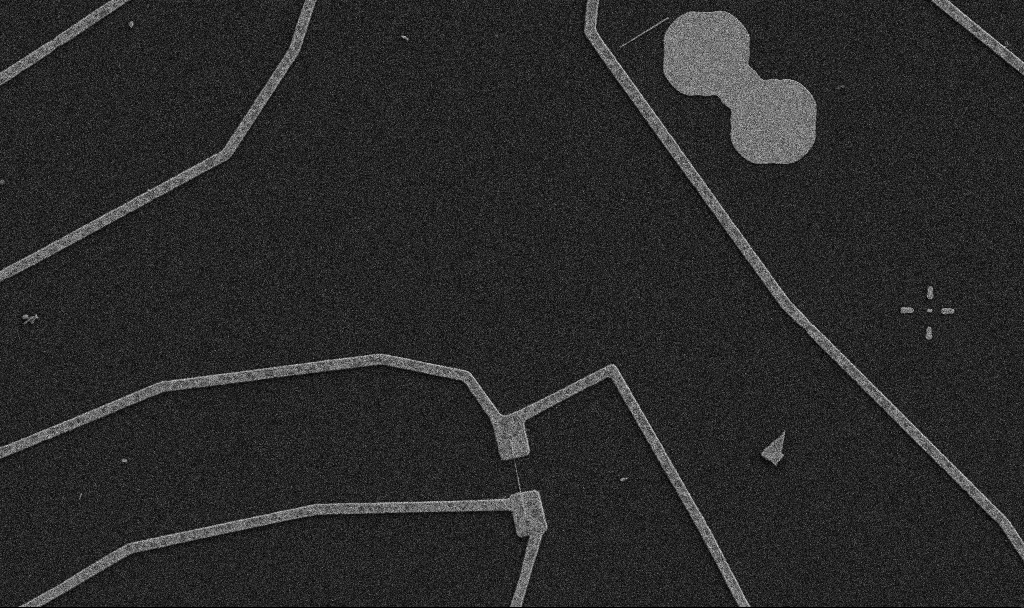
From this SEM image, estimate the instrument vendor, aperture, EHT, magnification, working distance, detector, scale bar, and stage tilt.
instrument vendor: Zeiss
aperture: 30 µm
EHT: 5 kV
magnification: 5 K X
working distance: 10.7 mm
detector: SE2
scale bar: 10000 nm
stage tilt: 0°